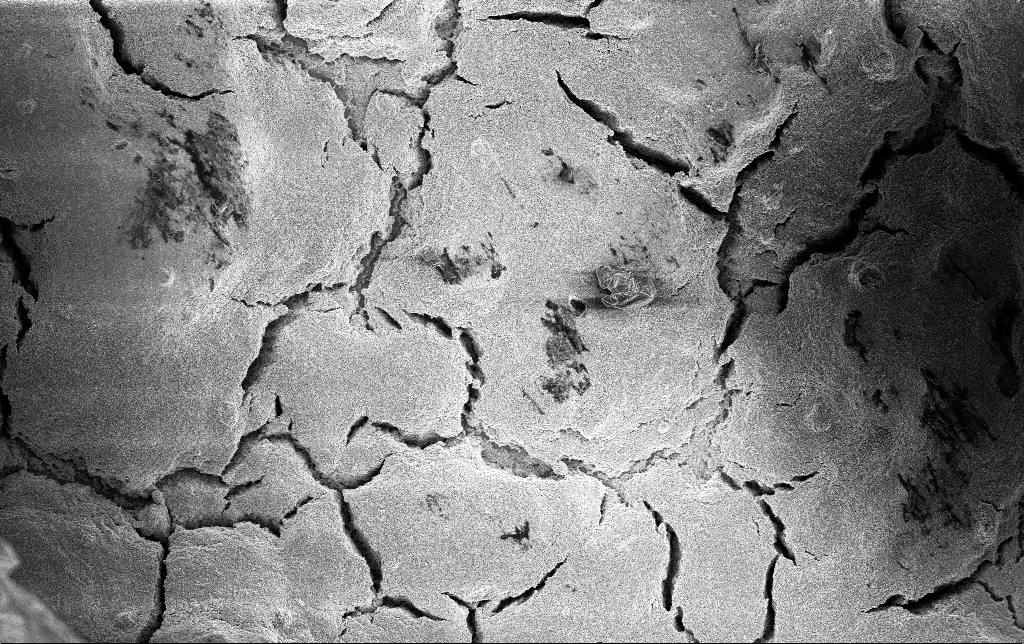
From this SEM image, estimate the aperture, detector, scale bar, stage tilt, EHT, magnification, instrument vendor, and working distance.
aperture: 30 µm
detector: InLens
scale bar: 10000 nm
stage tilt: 0°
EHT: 3 kV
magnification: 1.5 K X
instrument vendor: Zeiss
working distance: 2.8 mm